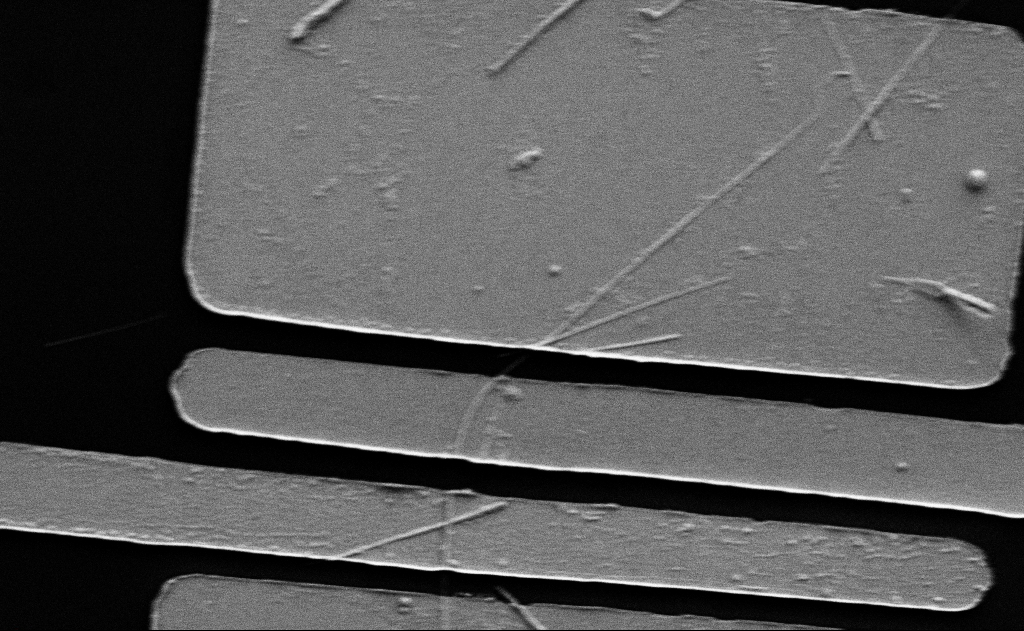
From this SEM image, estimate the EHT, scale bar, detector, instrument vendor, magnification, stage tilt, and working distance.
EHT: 5 kV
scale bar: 2000 nm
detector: SE2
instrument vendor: Zeiss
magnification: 10 K X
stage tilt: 0°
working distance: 15 mm